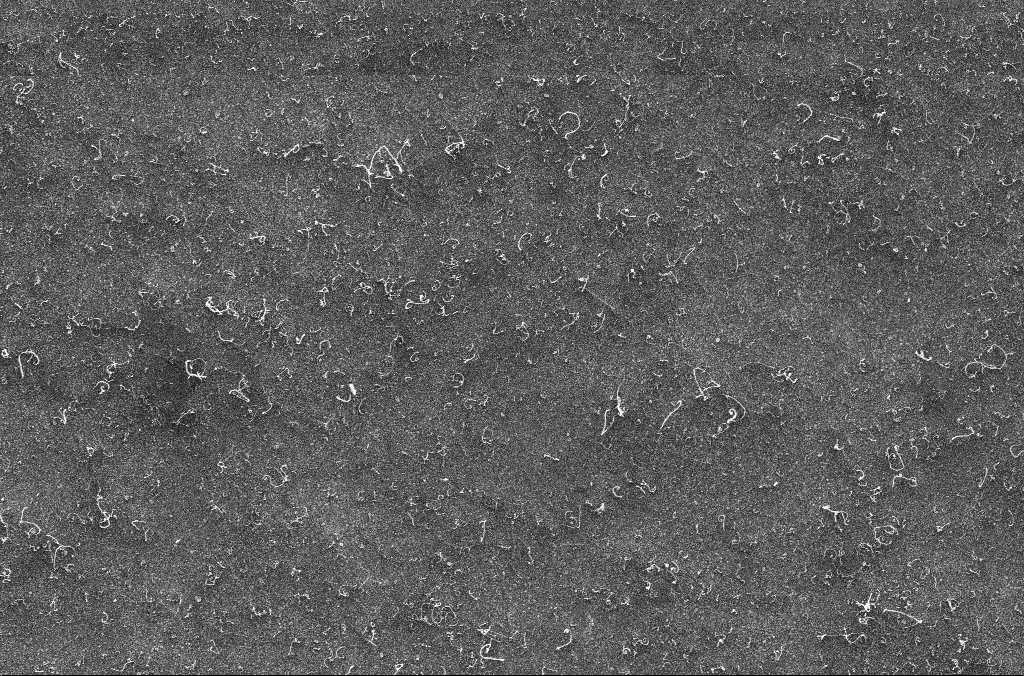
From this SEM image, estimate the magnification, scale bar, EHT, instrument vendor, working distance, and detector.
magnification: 22.38 K X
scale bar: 2000 nm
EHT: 10 kV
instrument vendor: Zeiss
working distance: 3.2 mm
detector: InLens